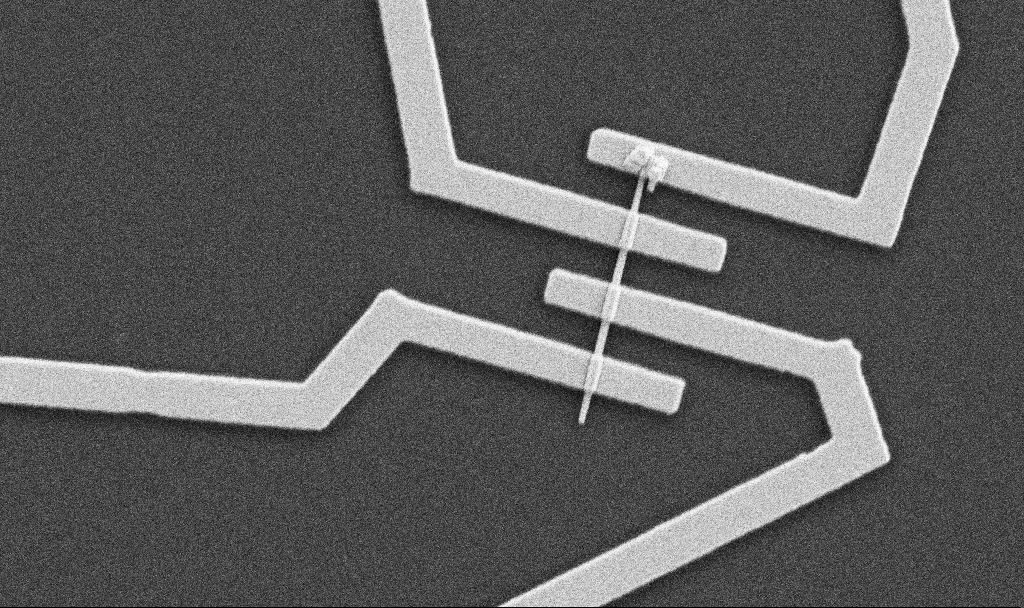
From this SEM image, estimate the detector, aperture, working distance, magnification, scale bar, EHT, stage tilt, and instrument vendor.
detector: SE2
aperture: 30 µm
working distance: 10.7 mm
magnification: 22.94 K X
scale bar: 2000 nm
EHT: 5 kV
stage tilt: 0°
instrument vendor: Zeiss